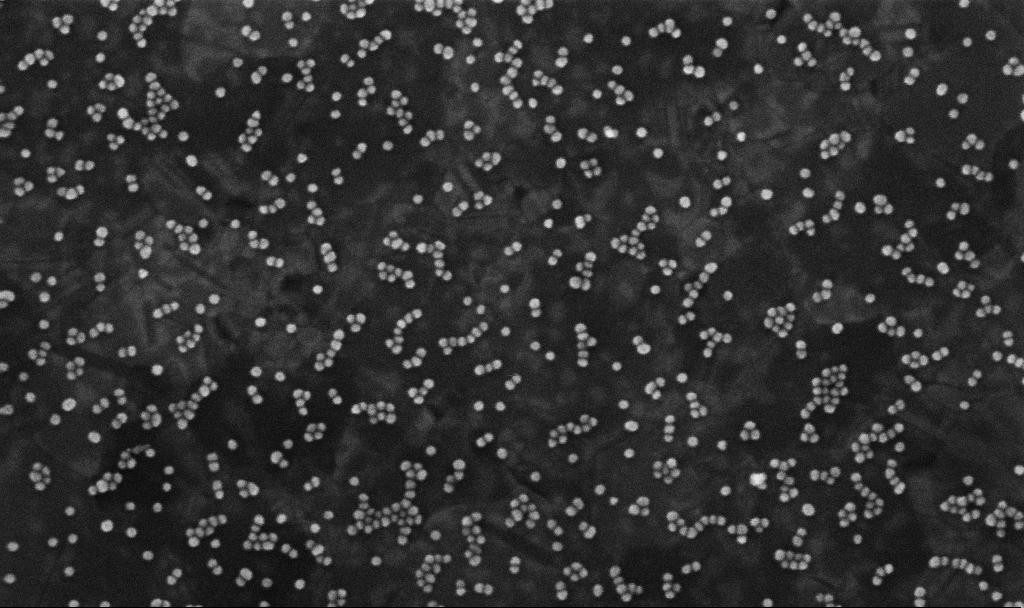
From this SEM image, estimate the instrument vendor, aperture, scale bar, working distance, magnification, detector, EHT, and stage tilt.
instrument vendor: Zeiss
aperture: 30 µm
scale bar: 100 nm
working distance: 3.8 mm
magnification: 200 K X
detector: InLens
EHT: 10 kV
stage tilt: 0°